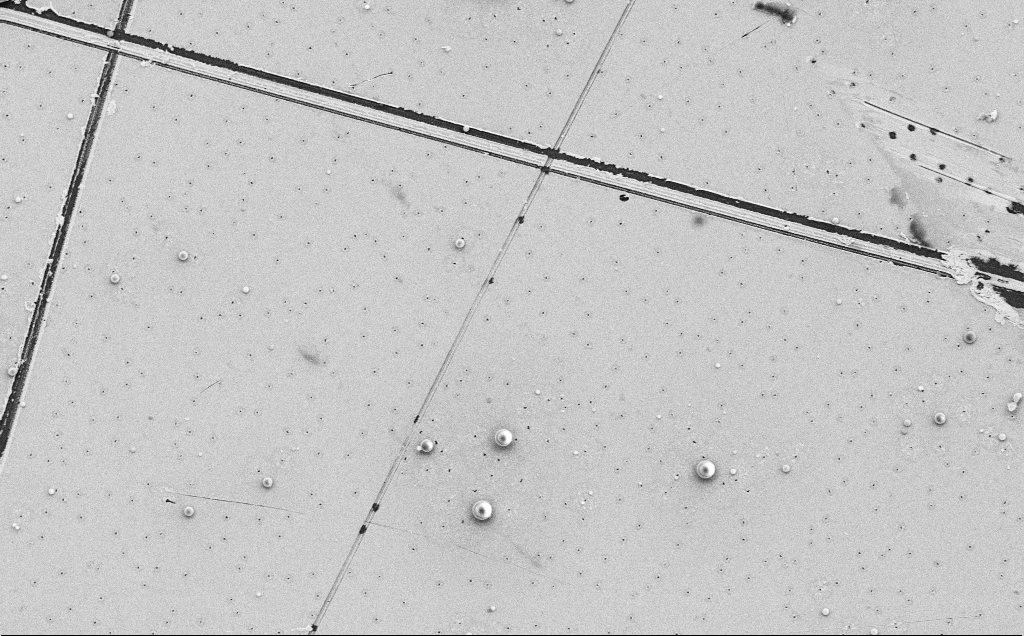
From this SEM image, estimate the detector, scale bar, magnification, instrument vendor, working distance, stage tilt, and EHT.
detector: SE2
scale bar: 10000 nm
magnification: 4.2 K X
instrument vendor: Zeiss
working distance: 12 mm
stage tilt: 0°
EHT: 5 kV